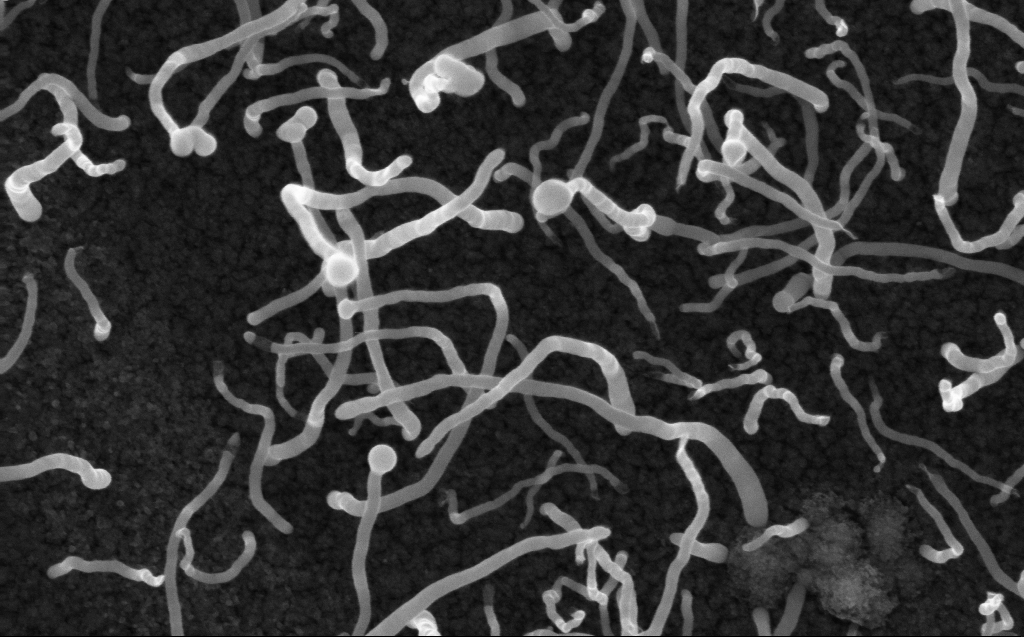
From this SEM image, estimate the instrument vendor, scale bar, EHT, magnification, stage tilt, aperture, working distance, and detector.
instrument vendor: Zeiss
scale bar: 200 nm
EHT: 10 kV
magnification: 100 K X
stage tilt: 0°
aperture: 30 µm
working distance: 3 mm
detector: InLens